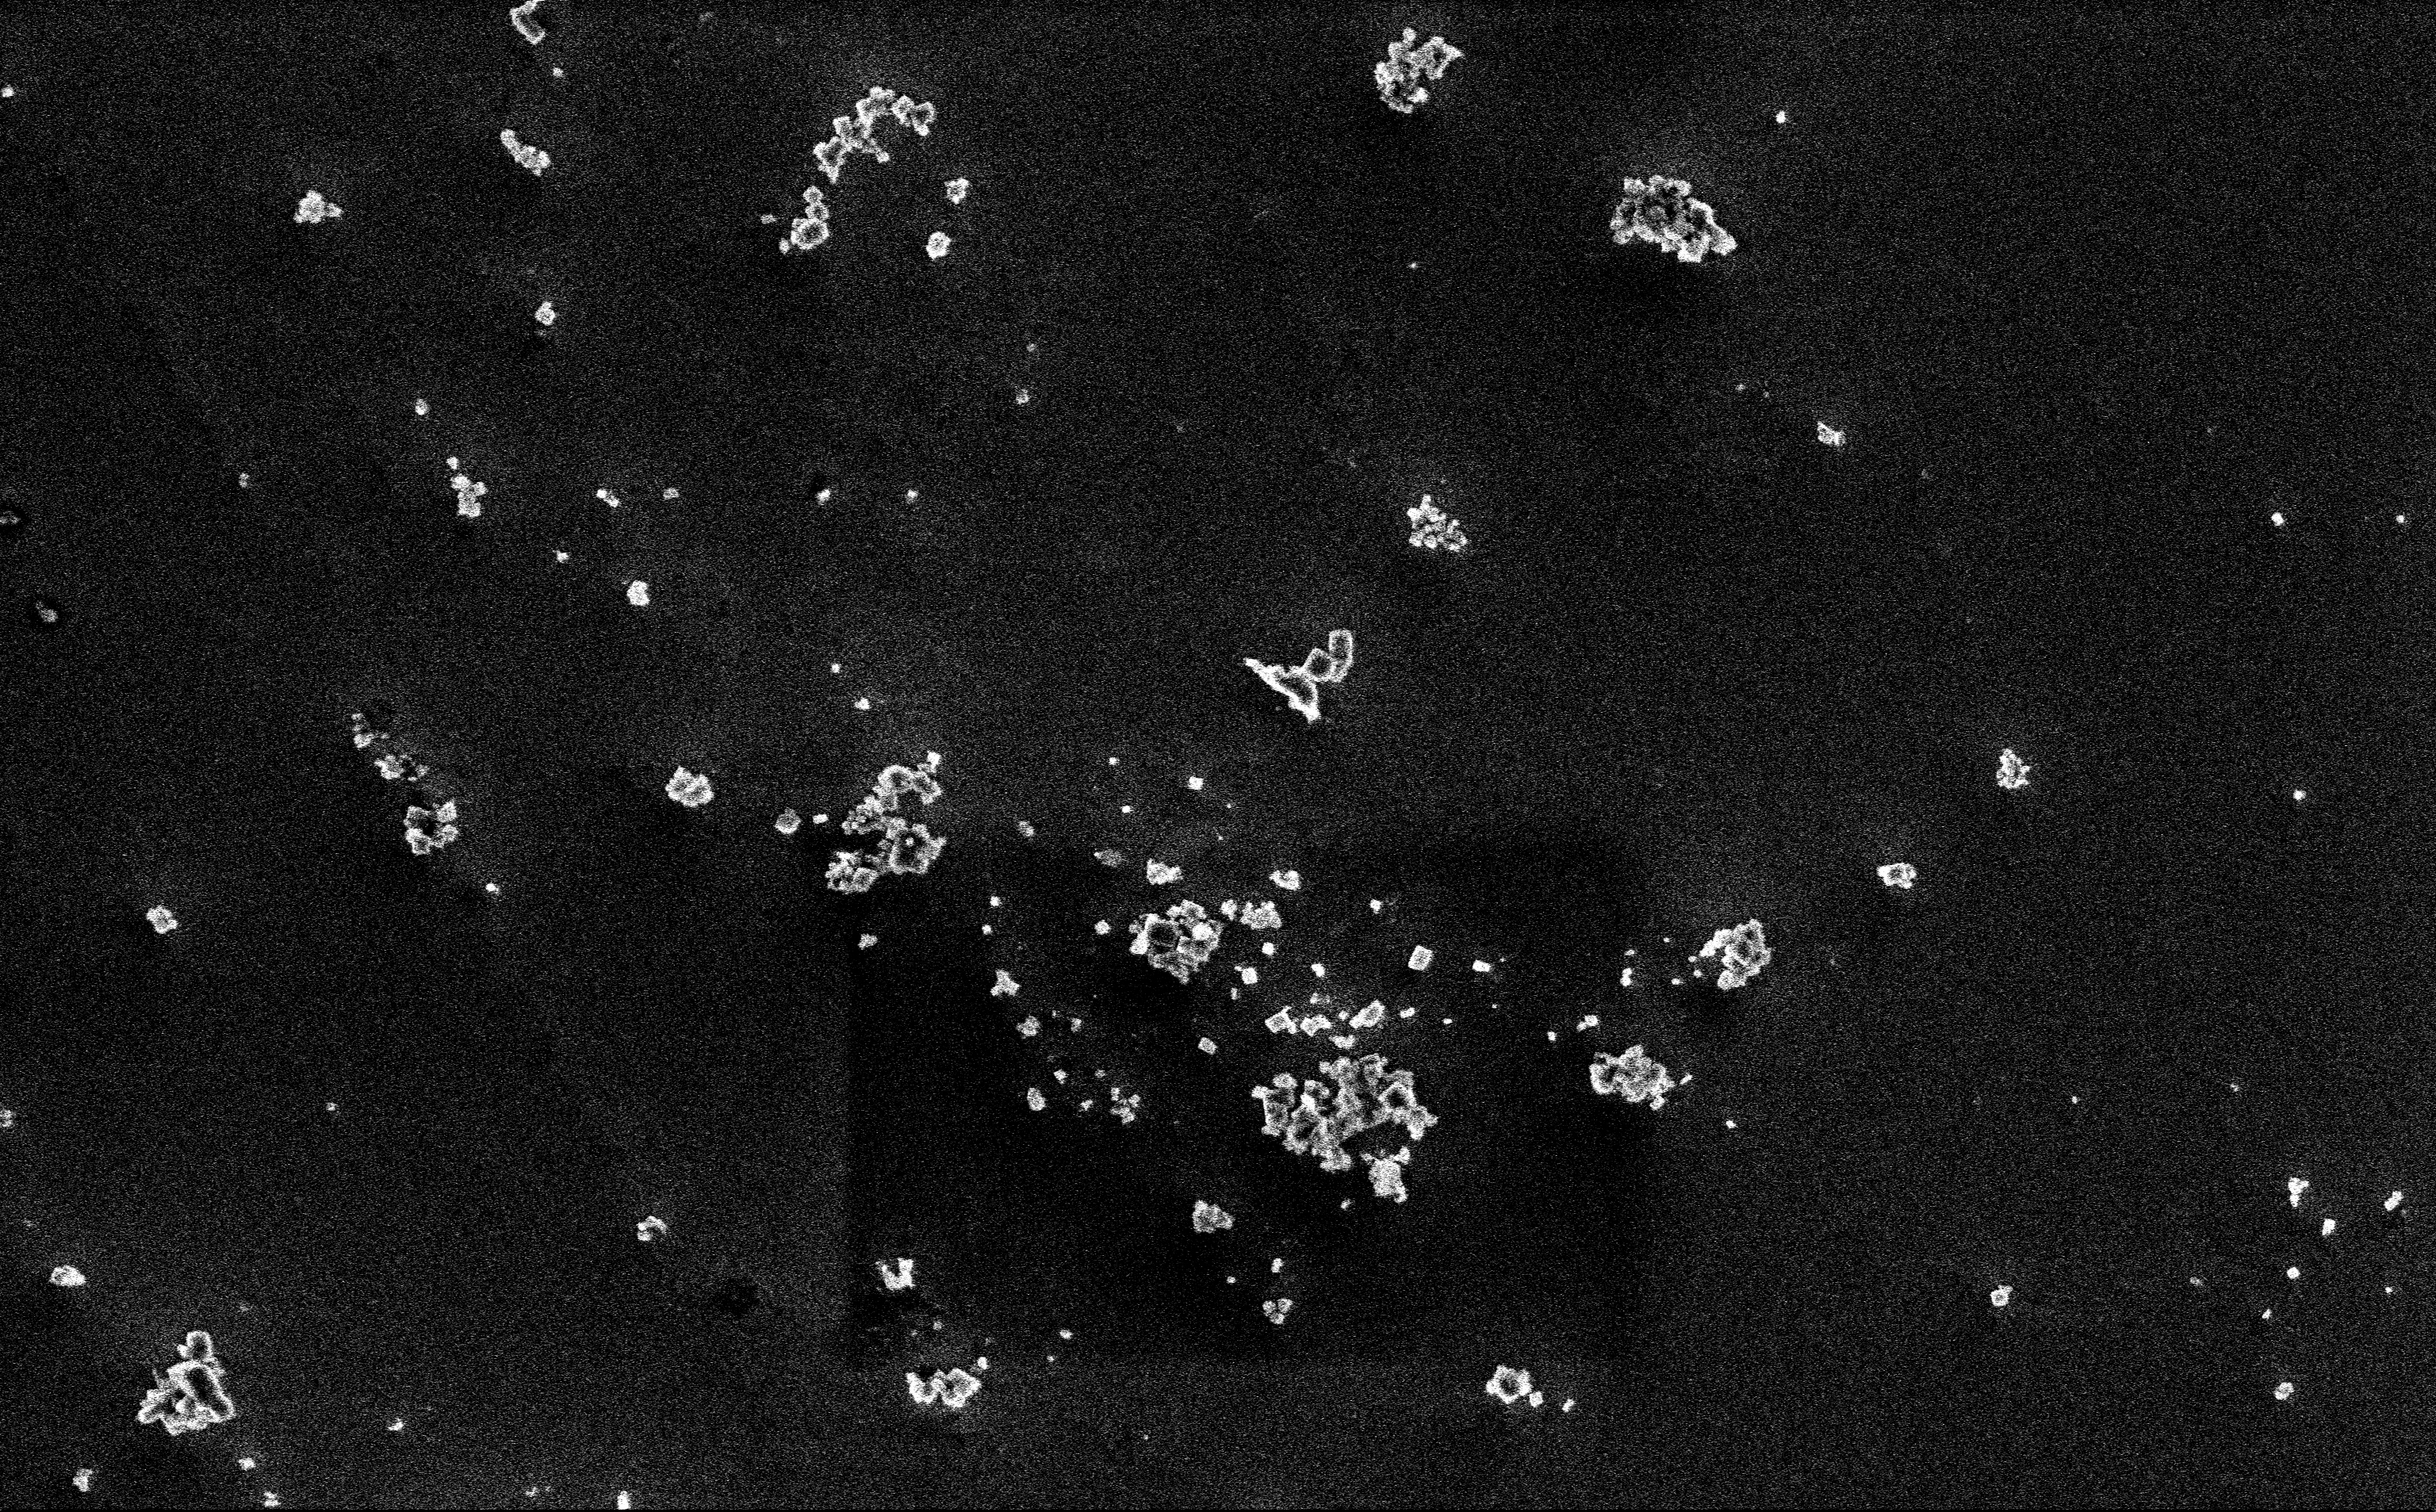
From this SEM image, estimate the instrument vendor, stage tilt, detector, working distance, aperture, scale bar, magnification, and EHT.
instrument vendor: Zeiss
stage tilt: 0°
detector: InLens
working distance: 3 mm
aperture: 30 µm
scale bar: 2000 nm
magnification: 12.85 K X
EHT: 3 kV